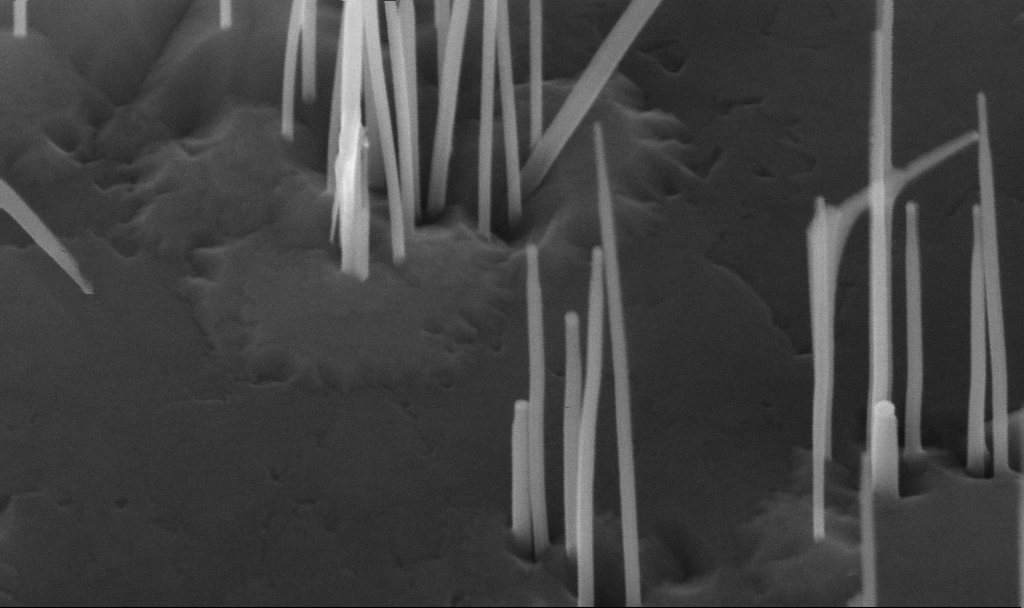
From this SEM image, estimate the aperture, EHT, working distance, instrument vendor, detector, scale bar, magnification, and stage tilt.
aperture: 30 µm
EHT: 10 kV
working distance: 5.6 mm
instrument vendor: Zeiss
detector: InLens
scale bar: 200 nm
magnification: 197.93 K X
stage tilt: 45°